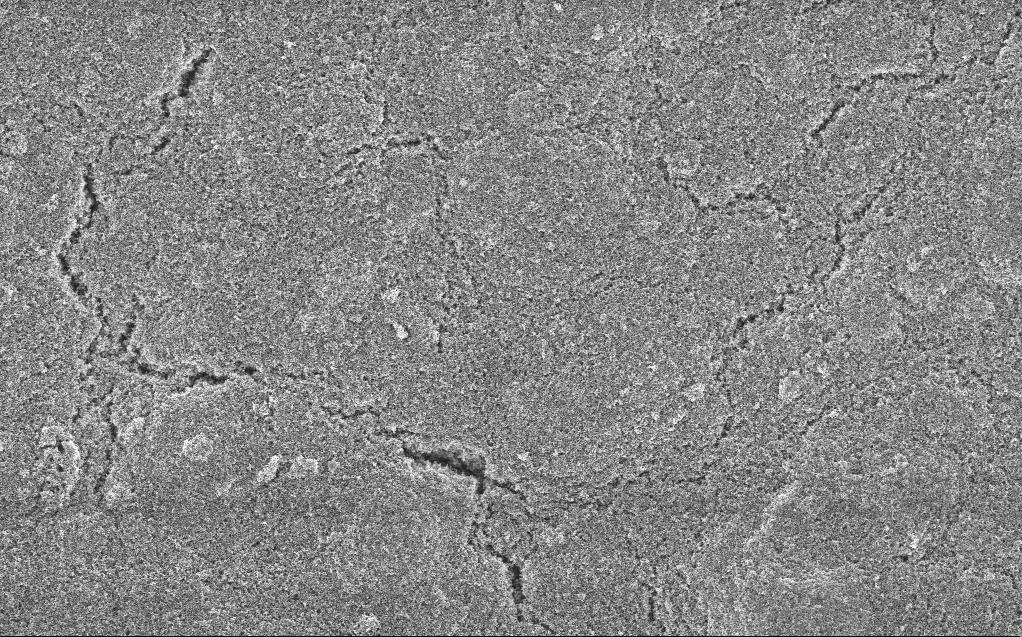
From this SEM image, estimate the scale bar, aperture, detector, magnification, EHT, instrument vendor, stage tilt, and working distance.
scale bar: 10000 nm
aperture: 30 µm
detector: InLens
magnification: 6.61 K X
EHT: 5 kV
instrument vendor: Zeiss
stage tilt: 0°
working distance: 4.4 mm